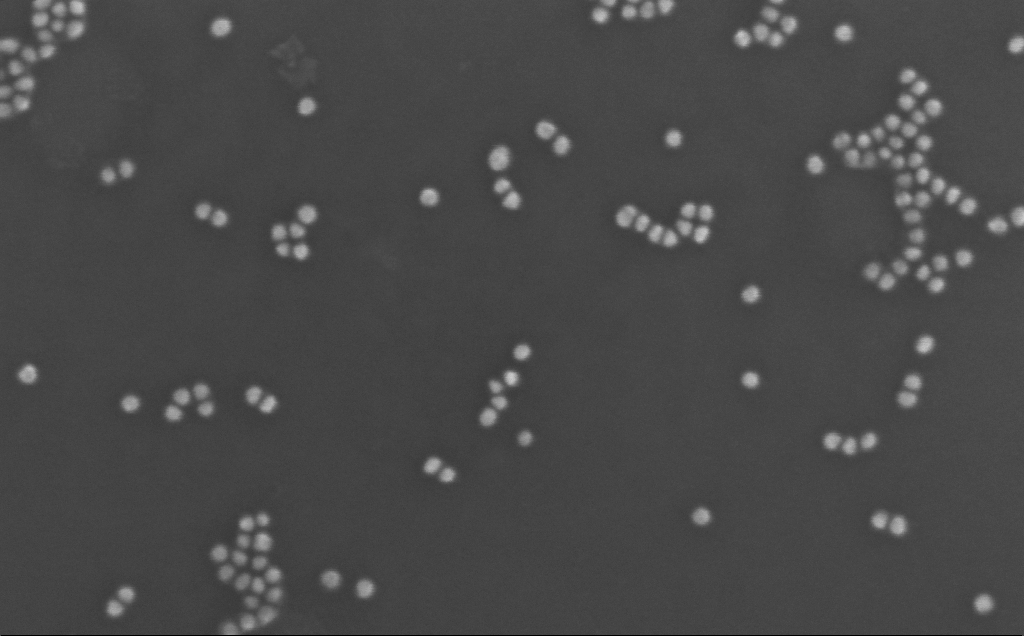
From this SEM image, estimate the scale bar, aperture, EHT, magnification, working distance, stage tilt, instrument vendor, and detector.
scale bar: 100 nm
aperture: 30 µm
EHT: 10 kV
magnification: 364.48 K X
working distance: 3 mm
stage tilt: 0°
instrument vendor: Zeiss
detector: InLens